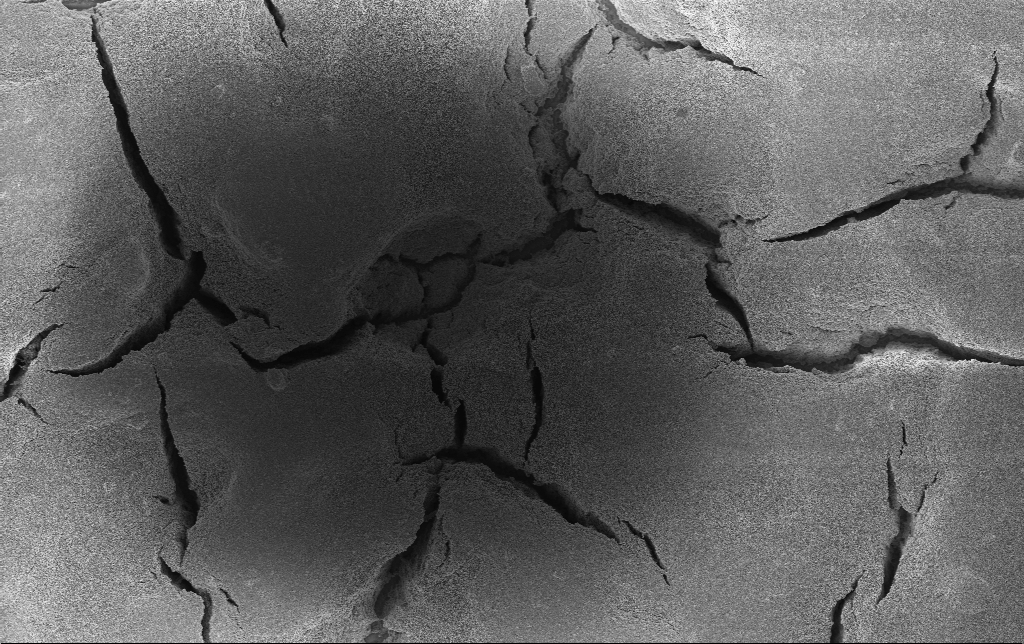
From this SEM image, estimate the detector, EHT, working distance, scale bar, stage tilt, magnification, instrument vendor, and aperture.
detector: InLens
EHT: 3 kV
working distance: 2.4 mm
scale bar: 20000 nm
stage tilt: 0°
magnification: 1.5 K X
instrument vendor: Zeiss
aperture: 30 µm